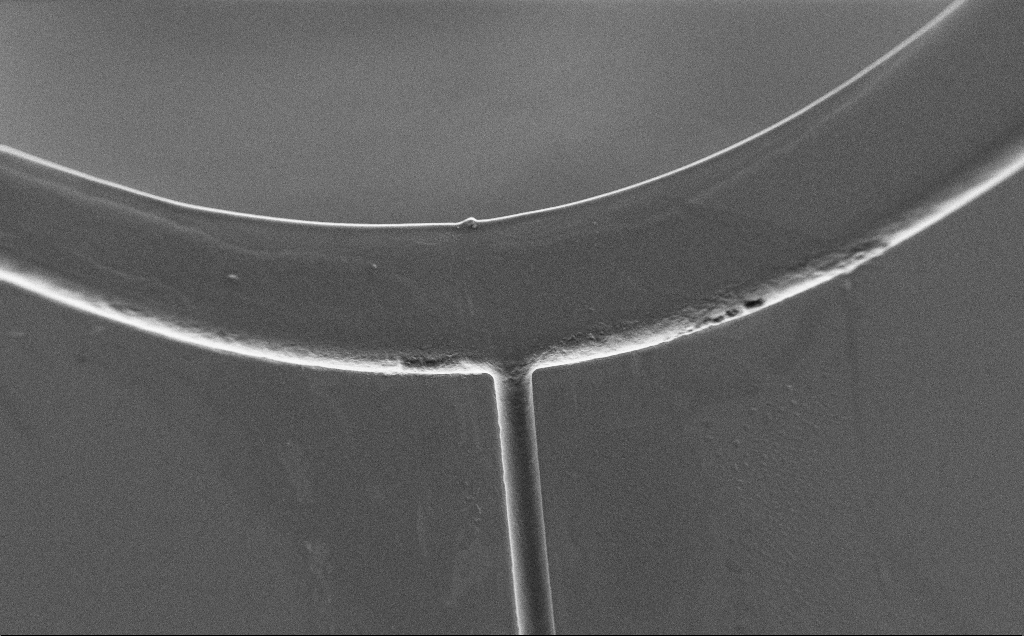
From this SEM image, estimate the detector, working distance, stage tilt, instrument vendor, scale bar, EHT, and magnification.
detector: SE2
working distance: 6 mm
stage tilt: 0°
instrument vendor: Zeiss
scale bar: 100000 nm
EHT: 0.8 kV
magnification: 0.308 K X